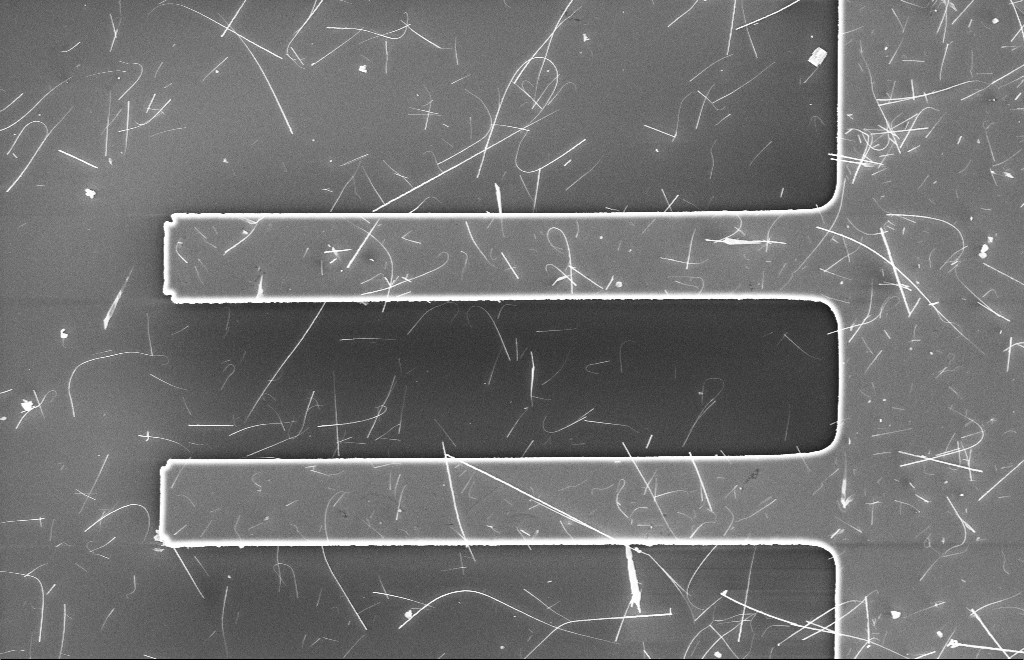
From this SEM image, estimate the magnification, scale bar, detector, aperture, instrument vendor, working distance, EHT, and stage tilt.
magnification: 2.5 K X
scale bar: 10000 nm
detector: InLens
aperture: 20 µm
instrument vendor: Zeiss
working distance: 6 mm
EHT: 10 kV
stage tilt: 0°